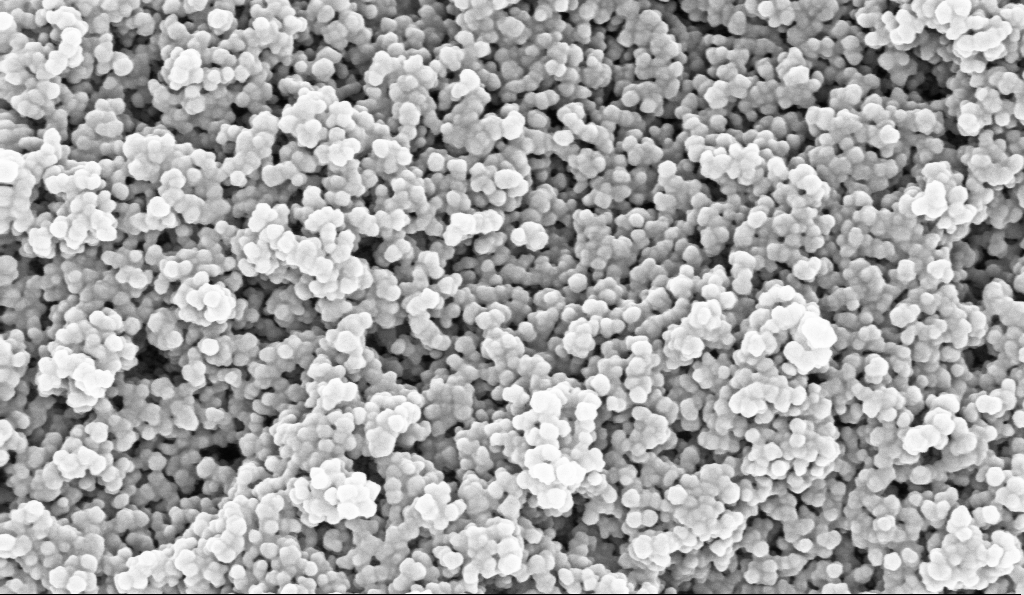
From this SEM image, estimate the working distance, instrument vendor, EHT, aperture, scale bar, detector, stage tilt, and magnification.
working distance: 5.1 mm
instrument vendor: Zeiss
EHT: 10 kV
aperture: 30 µm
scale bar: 200 nm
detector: InLens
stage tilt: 0°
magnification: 135 K X